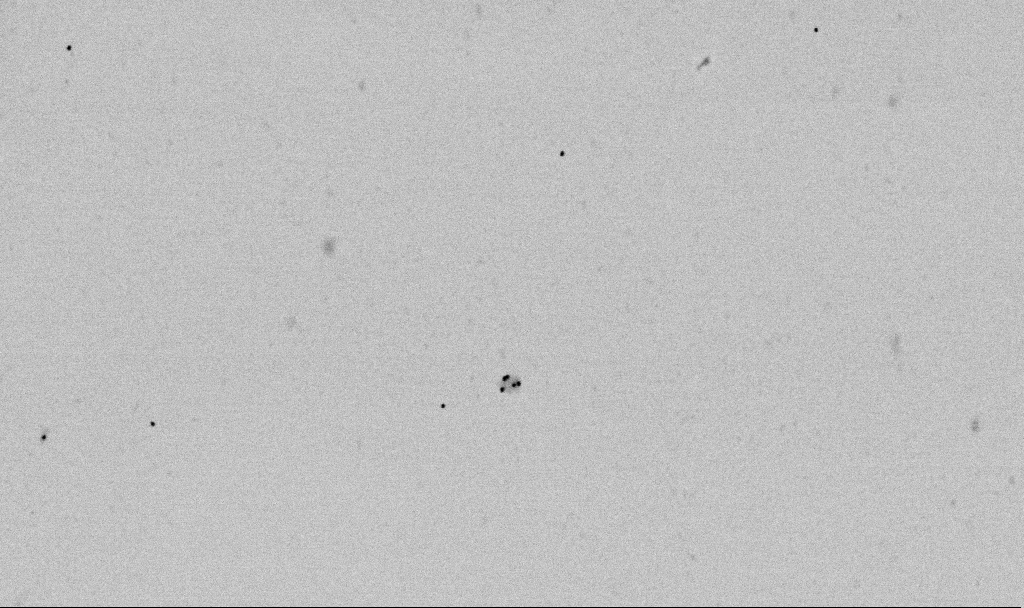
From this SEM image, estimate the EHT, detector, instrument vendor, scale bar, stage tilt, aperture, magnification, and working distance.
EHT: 2 kV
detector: SE2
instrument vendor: Zeiss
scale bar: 1000 nm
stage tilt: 0°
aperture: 30 µm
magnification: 50 K X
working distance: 6.5 mm